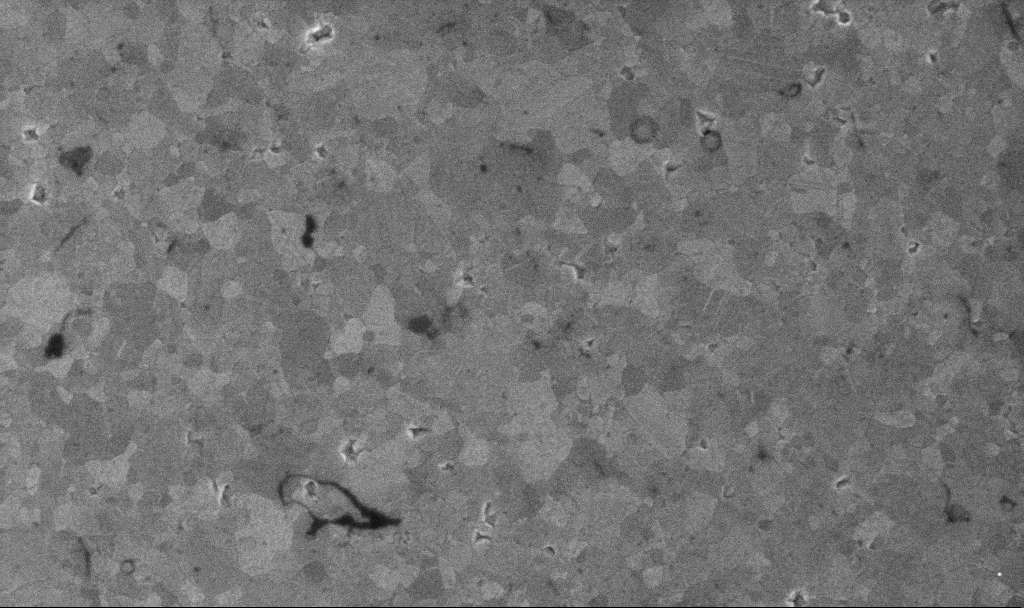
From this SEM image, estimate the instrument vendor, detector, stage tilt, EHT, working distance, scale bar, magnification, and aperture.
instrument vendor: Zeiss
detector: InLens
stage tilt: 0°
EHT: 10 kV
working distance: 3.1 mm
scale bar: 1000 nm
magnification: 50.56 K X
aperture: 30 µm